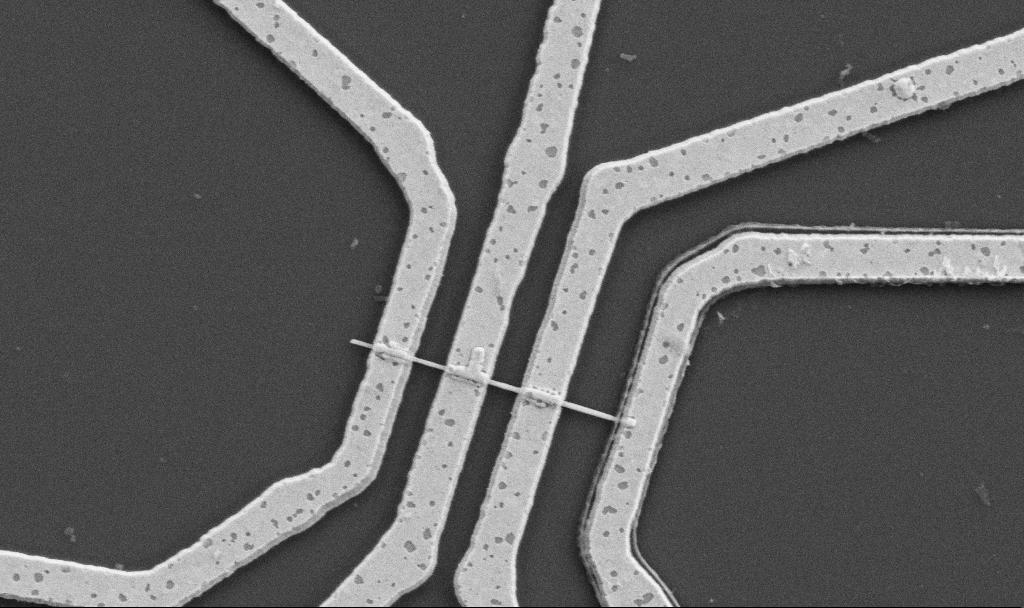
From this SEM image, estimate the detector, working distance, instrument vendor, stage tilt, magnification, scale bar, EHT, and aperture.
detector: SE2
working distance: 10.7 mm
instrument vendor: Zeiss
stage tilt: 0°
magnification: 20 K X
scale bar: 1000 nm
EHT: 5 kV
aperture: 30 µm